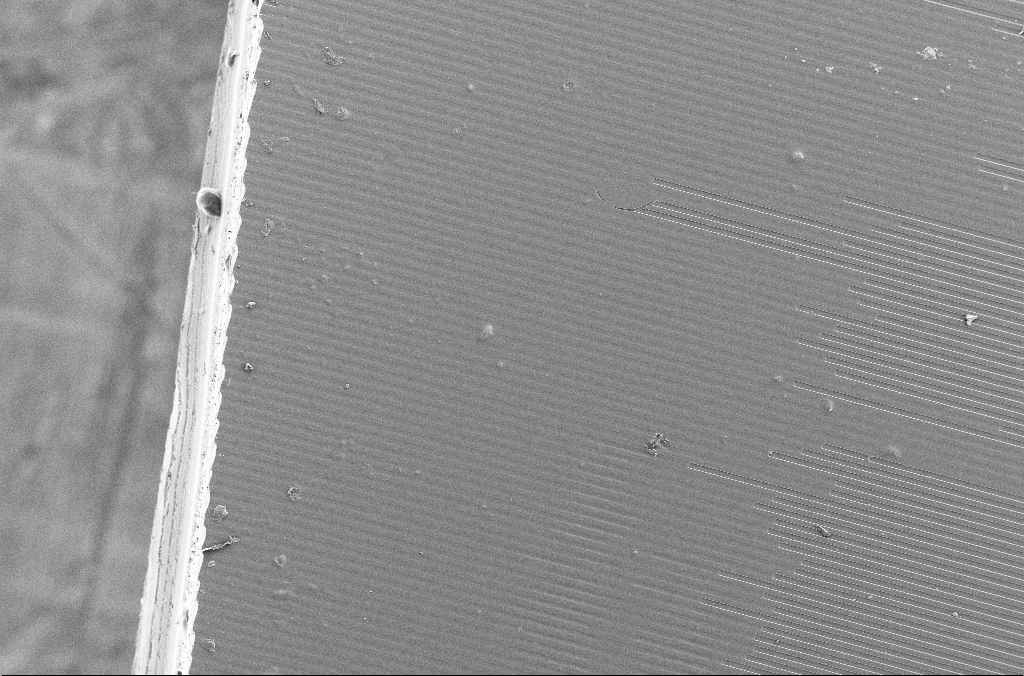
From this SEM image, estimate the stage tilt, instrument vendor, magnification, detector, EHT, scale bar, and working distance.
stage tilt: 0°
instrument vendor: Zeiss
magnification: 0.2 K X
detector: SE2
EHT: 5 kV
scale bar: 100000 nm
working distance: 4.1 mm